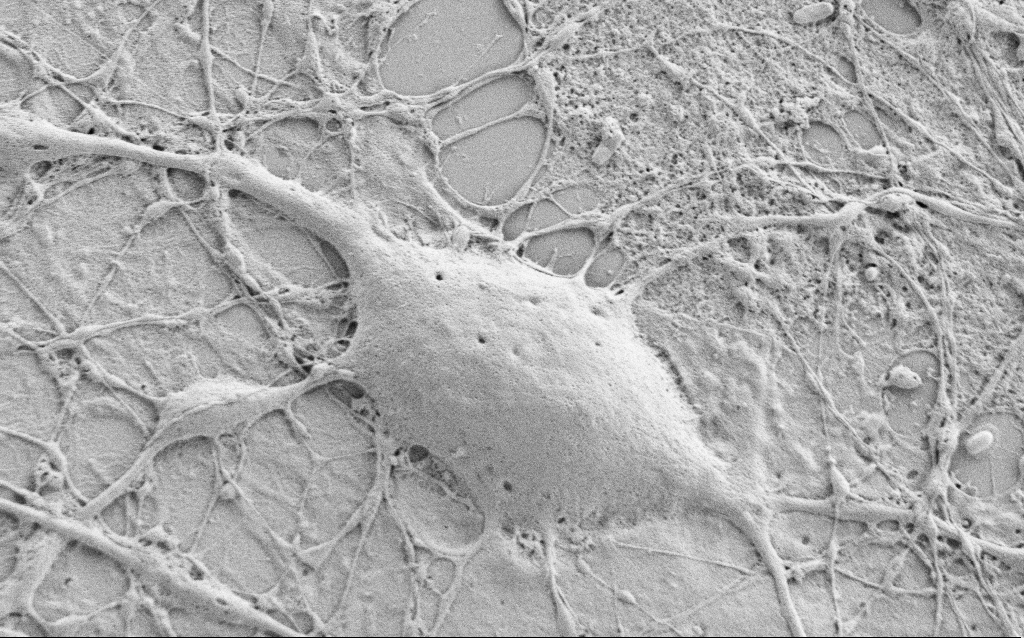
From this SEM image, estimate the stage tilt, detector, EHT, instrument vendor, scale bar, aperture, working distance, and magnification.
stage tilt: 0°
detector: SE2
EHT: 0.8 kV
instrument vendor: Zeiss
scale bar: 2000 nm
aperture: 30 µm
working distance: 5.8 mm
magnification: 7.5 K X